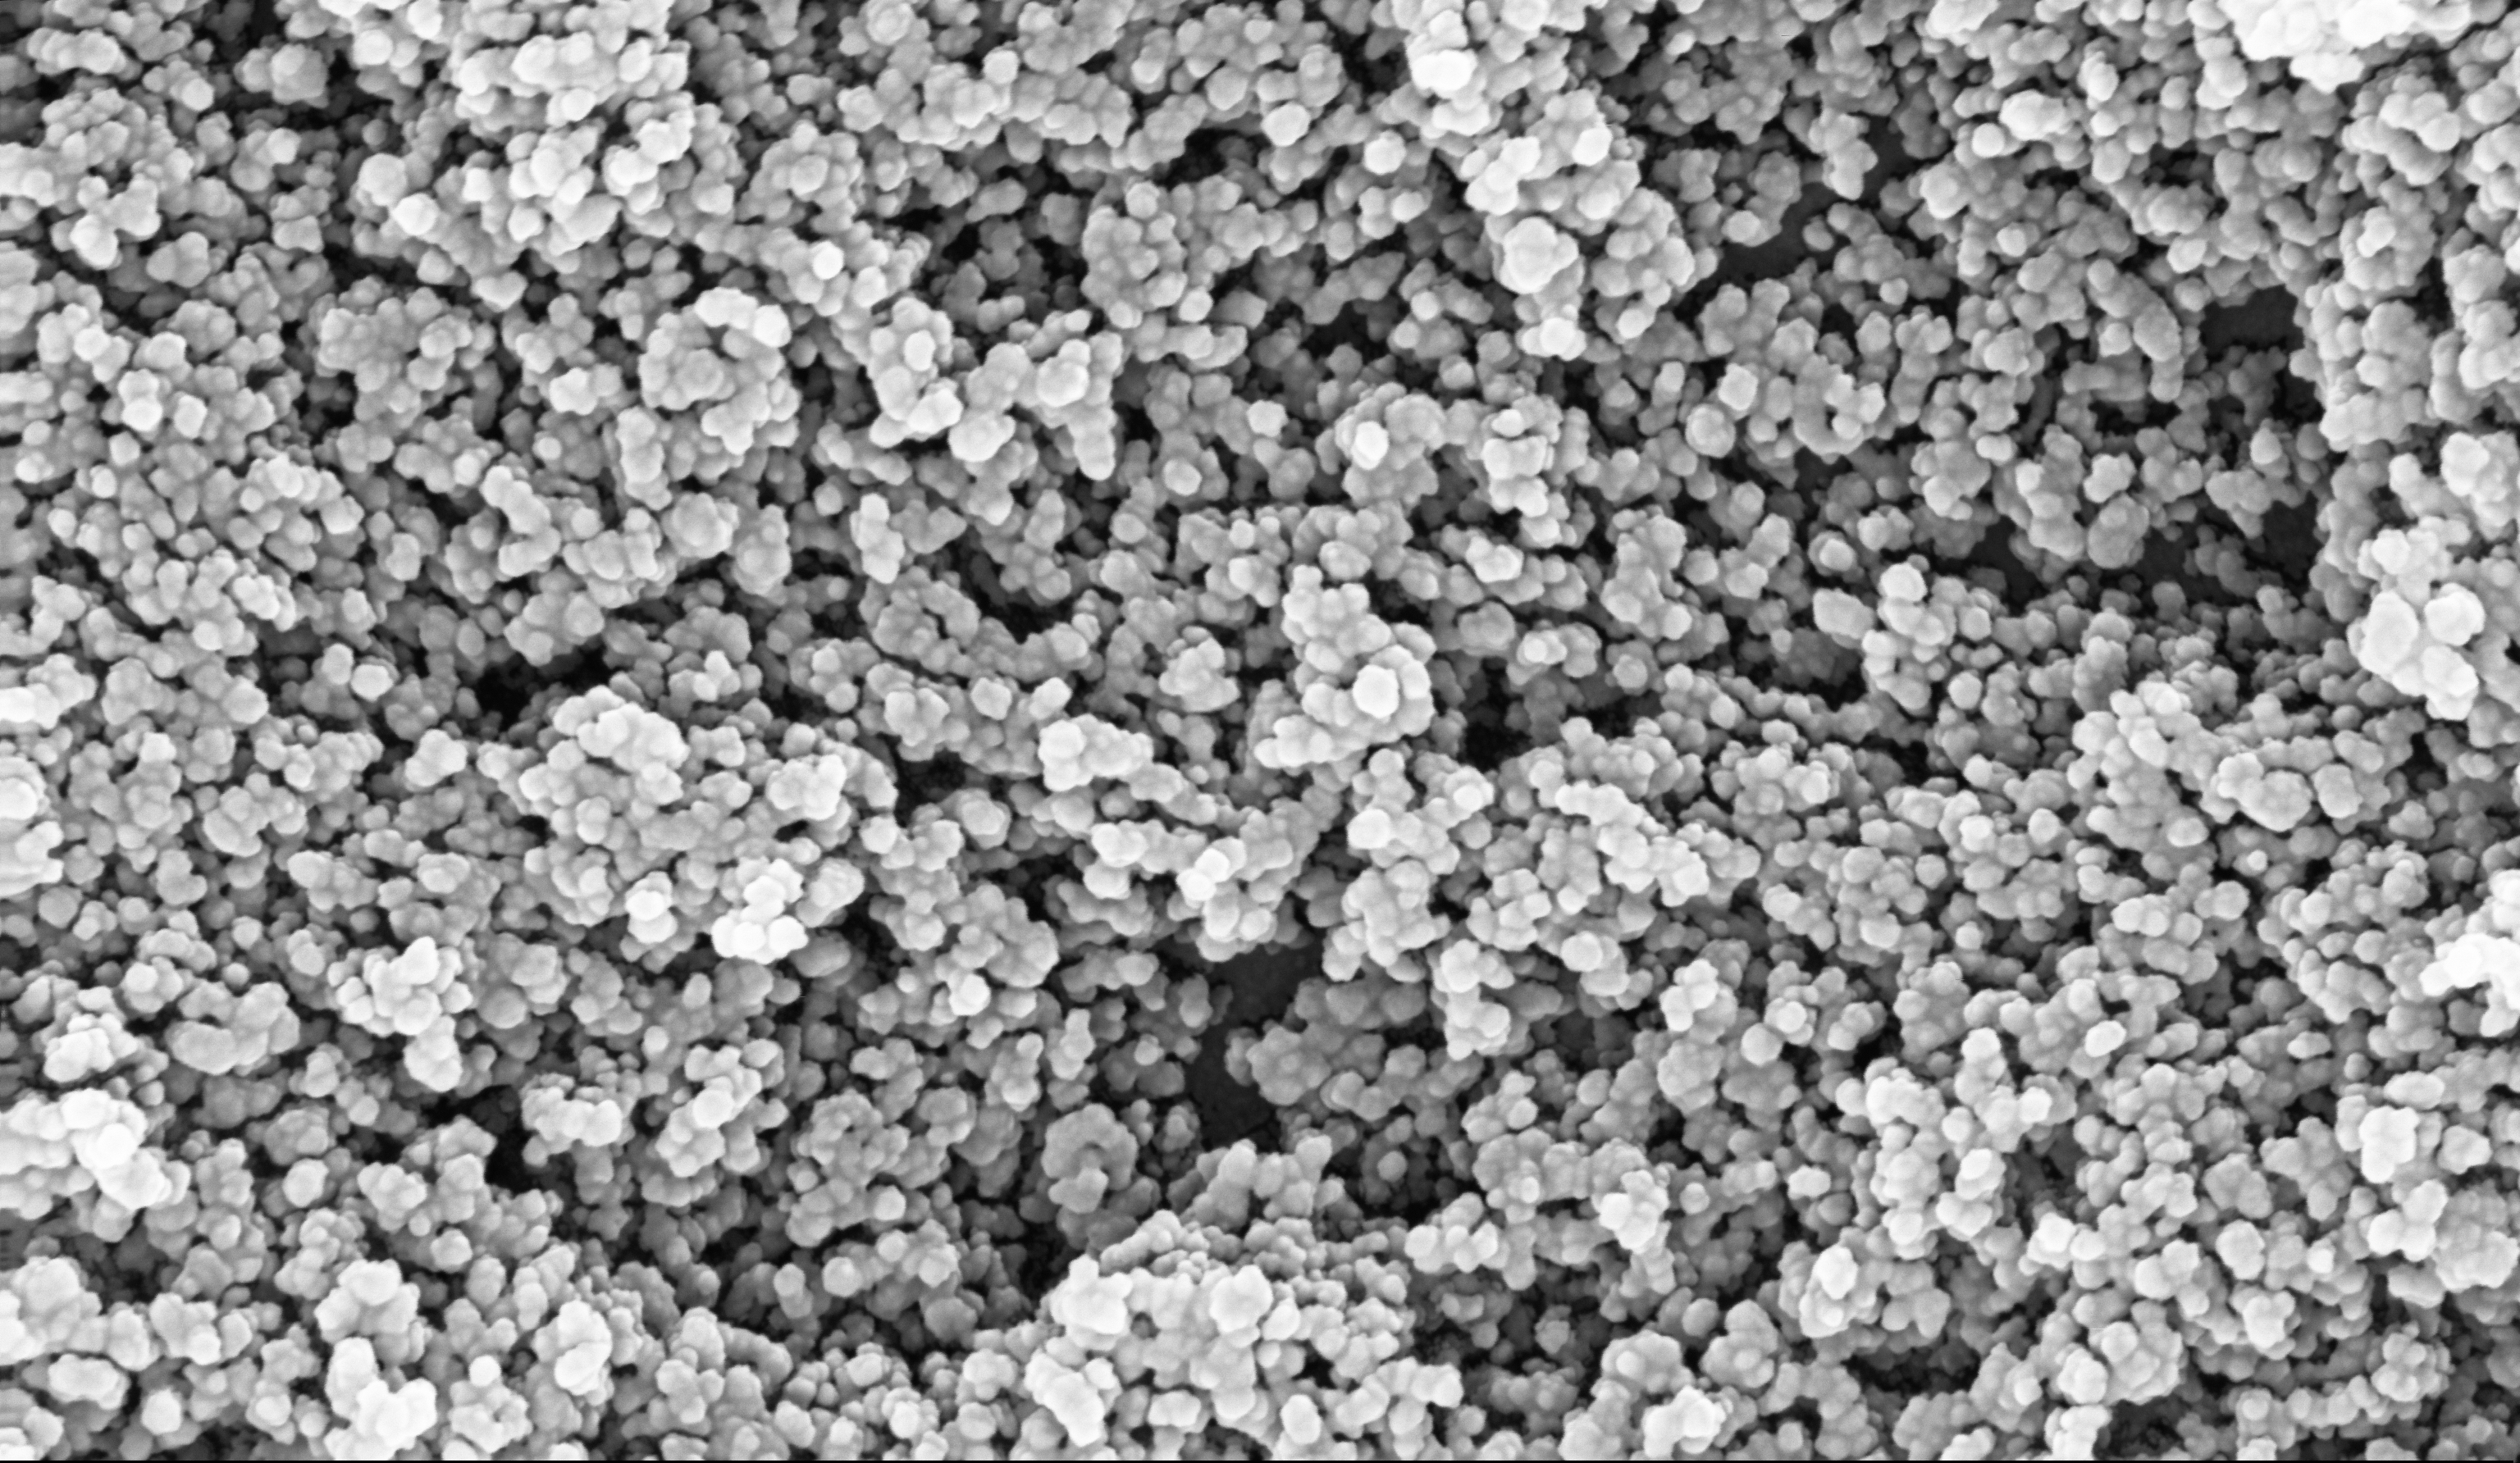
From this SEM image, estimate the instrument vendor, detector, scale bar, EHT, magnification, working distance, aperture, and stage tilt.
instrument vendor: Zeiss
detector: InLens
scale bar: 200 nm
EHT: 10 kV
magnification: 135 K X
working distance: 5.1 mm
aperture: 30 µm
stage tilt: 0°